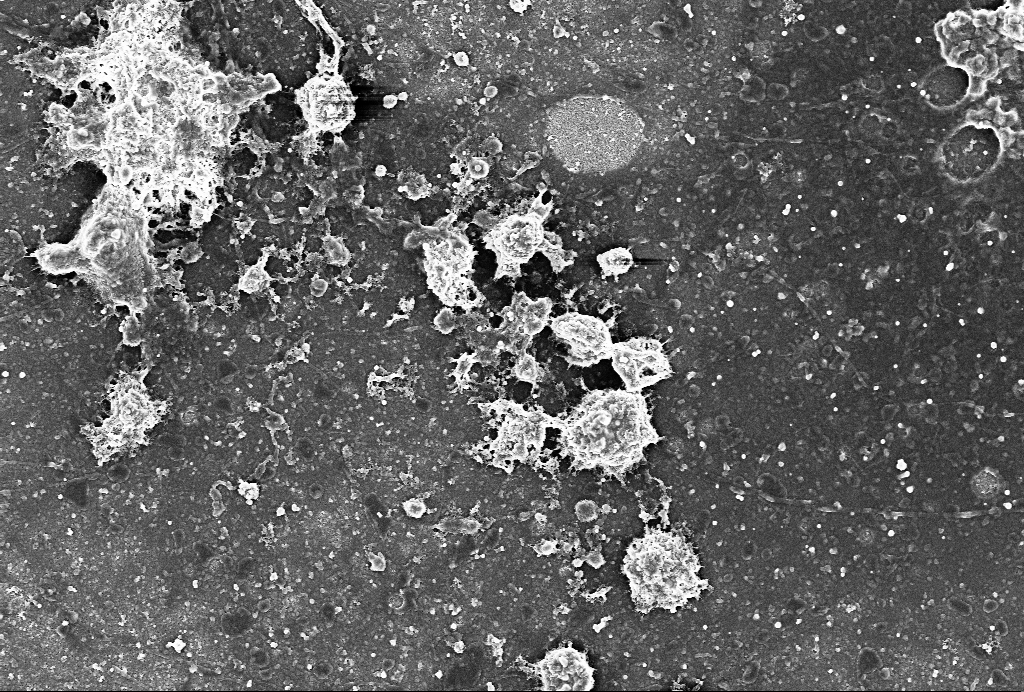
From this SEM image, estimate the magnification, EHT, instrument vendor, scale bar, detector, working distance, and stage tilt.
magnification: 5 K X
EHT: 4 kV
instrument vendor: Zeiss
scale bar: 10000 nm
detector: SE2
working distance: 6 mm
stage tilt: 0°